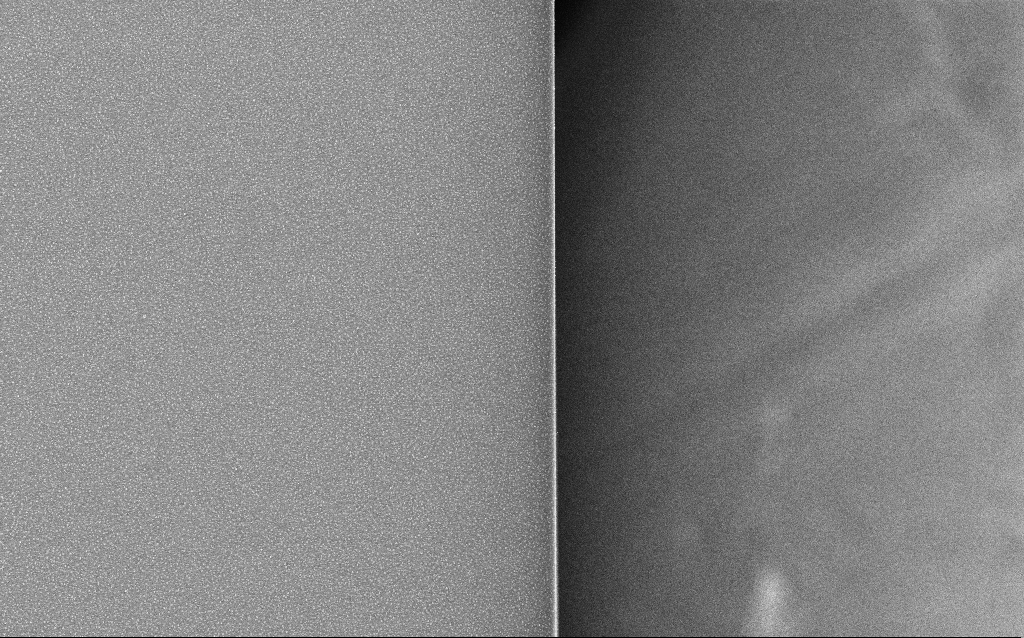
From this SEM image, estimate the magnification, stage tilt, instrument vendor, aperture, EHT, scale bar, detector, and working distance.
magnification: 1 K X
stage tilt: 0°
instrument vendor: Zeiss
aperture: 30 µm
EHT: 20 kV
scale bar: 20000 nm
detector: InLens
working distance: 1.8 mm